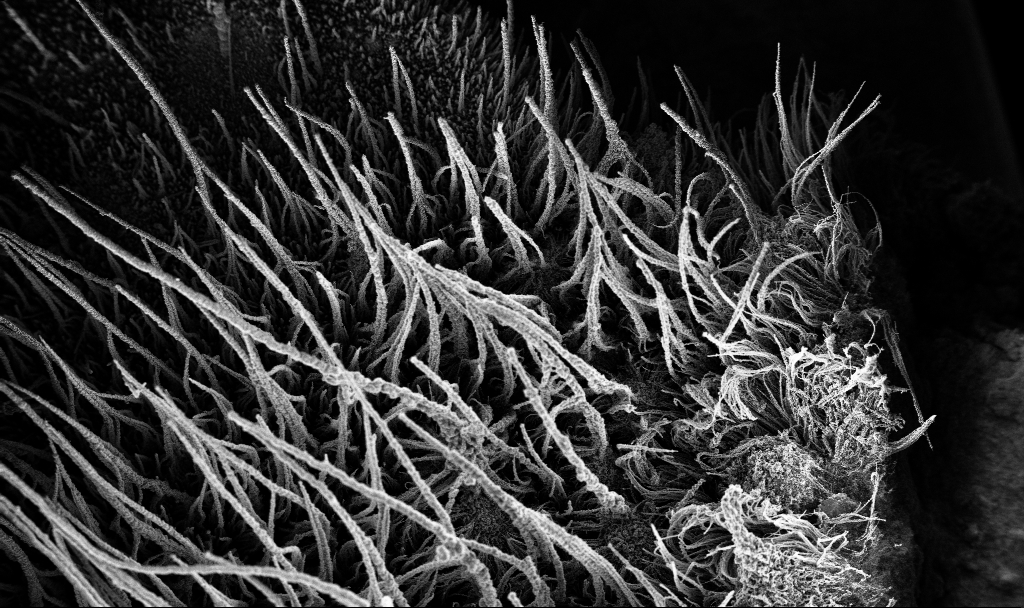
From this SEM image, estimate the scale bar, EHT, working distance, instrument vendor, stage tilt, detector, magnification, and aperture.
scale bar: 100000 nm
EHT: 3 kV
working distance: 3.8 mm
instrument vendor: Zeiss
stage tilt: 0°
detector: InLens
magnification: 0.15 K X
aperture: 30 µm